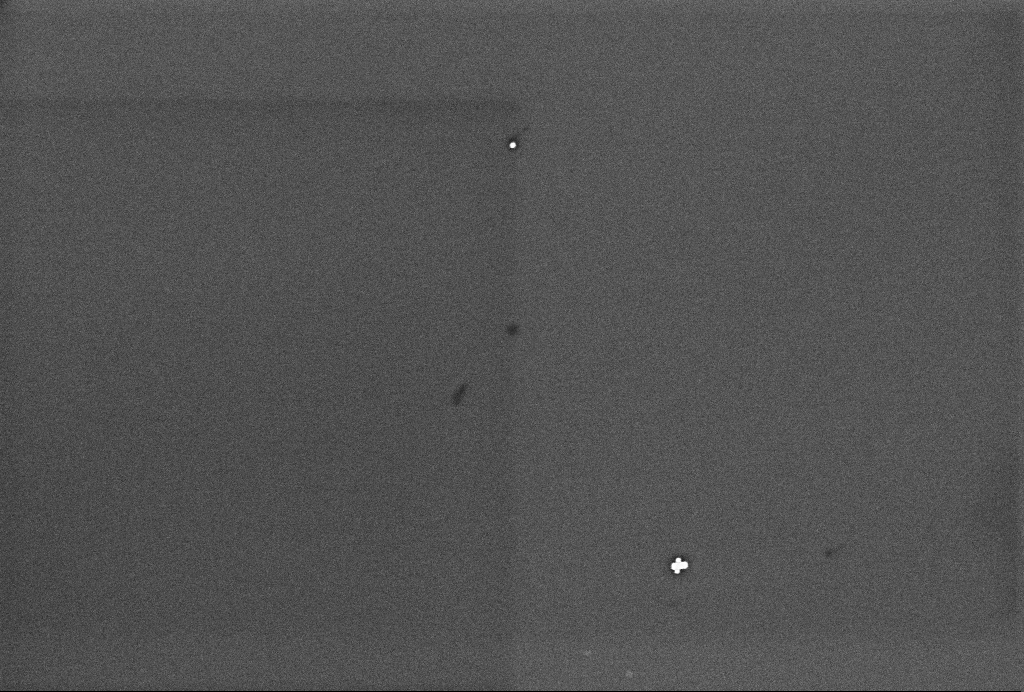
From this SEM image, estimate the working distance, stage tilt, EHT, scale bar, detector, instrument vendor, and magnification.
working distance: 3.3 mm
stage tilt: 0°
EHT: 2 kV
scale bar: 200 nm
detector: InLens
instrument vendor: Zeiss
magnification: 80.44 K X